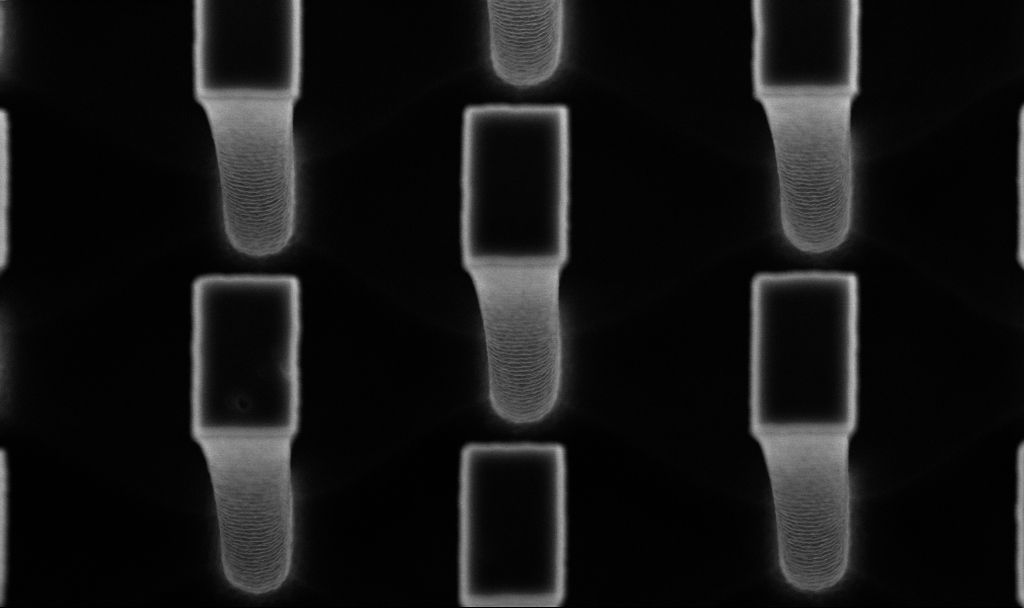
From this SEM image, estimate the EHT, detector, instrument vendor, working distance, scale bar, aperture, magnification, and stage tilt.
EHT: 5 kV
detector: InLens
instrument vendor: Zeiss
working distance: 4.8 mm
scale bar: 1000 nm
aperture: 30 µm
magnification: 25.85 K X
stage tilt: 10°